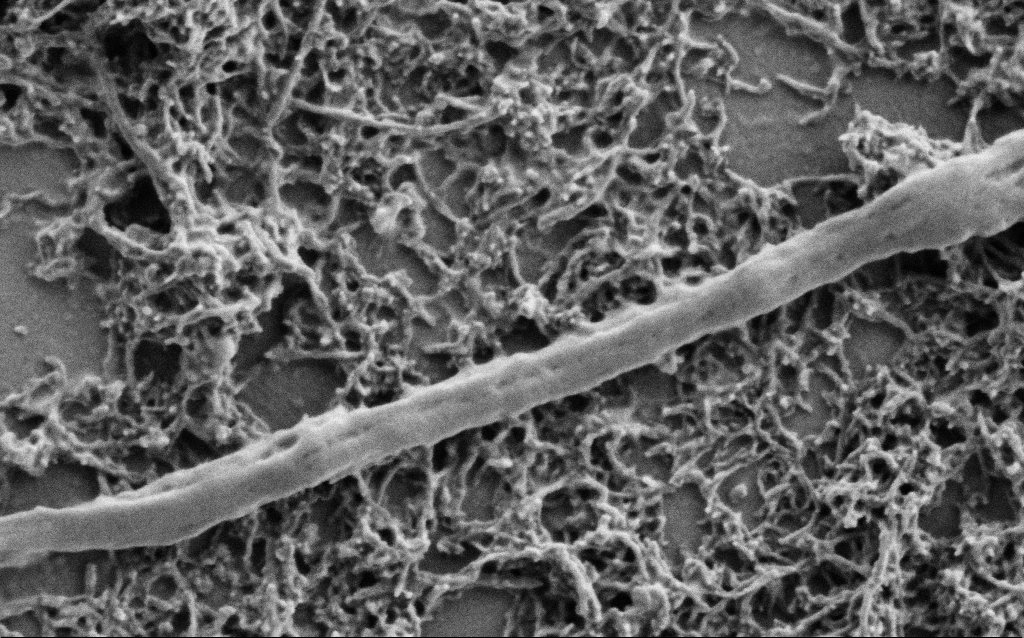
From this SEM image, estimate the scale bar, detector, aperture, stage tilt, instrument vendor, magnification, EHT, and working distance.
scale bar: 200 nm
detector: SE2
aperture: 30 µm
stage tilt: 0°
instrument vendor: Zeiss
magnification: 75 K X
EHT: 1 kV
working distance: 7 mm